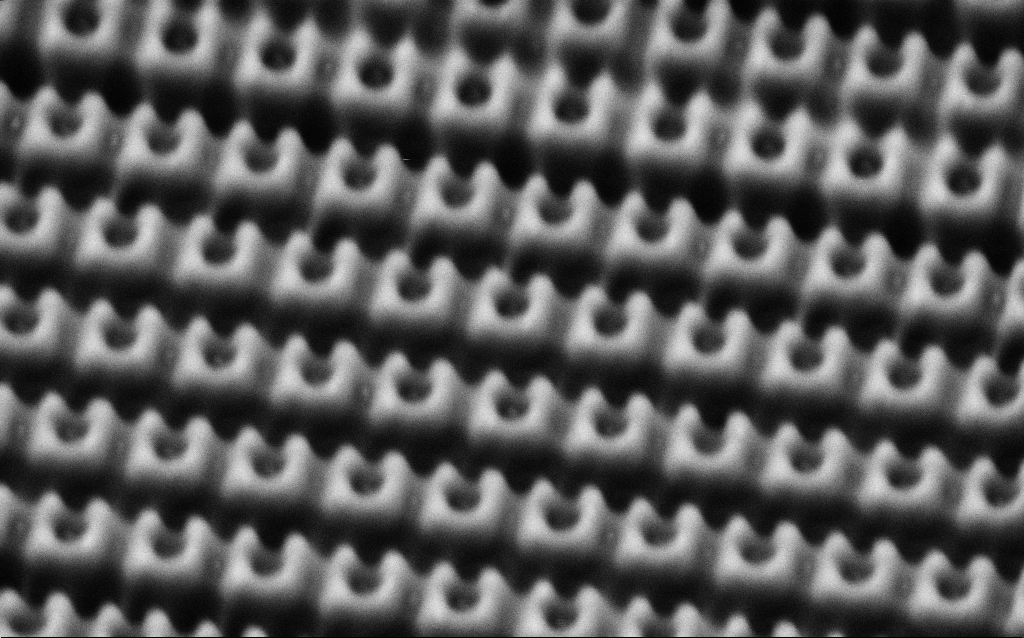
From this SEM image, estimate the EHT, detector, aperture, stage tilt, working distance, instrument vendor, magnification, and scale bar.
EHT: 1.5 kV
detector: SE2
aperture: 30 µm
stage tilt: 30°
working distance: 6.5 mm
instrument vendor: Zeiss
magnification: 79.01 K X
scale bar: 200 nm